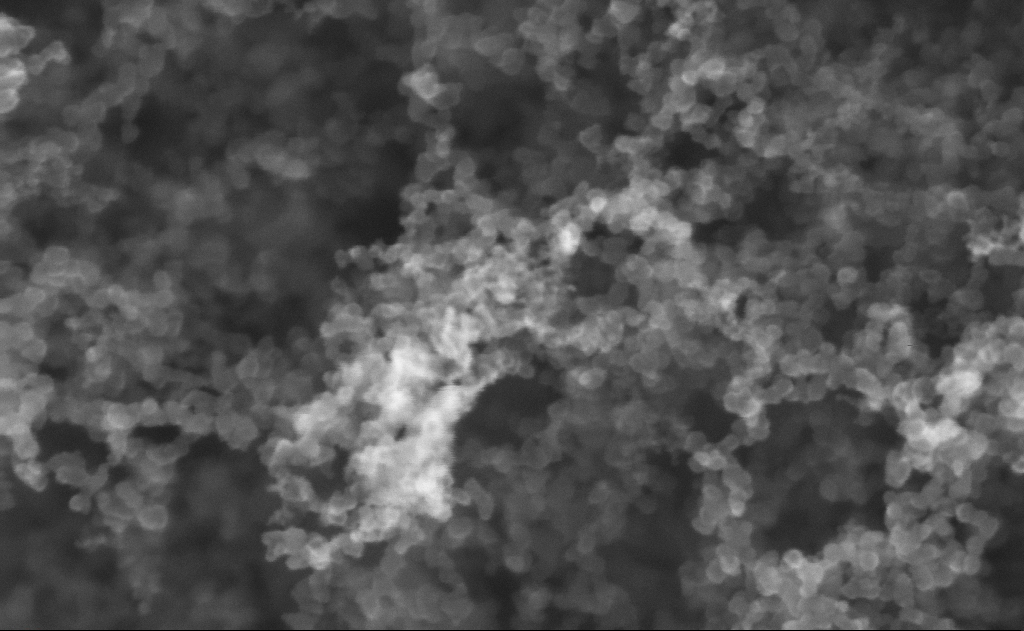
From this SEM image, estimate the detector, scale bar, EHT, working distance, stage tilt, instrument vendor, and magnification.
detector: InLens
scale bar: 100 nm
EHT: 10 kV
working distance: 2 mm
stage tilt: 0.6°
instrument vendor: Zeiss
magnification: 401.44 K X